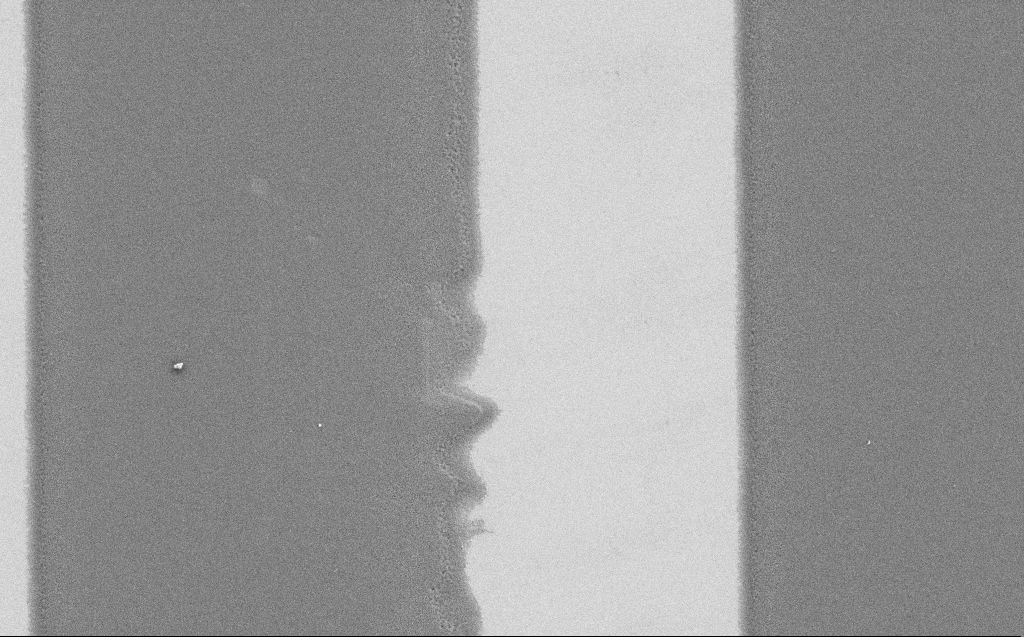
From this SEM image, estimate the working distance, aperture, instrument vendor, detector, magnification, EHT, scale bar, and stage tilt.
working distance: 6 mm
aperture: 30 µm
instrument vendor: Zeiss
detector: SE2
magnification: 9.42 K X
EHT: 1.2 kV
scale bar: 2000 nm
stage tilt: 0°